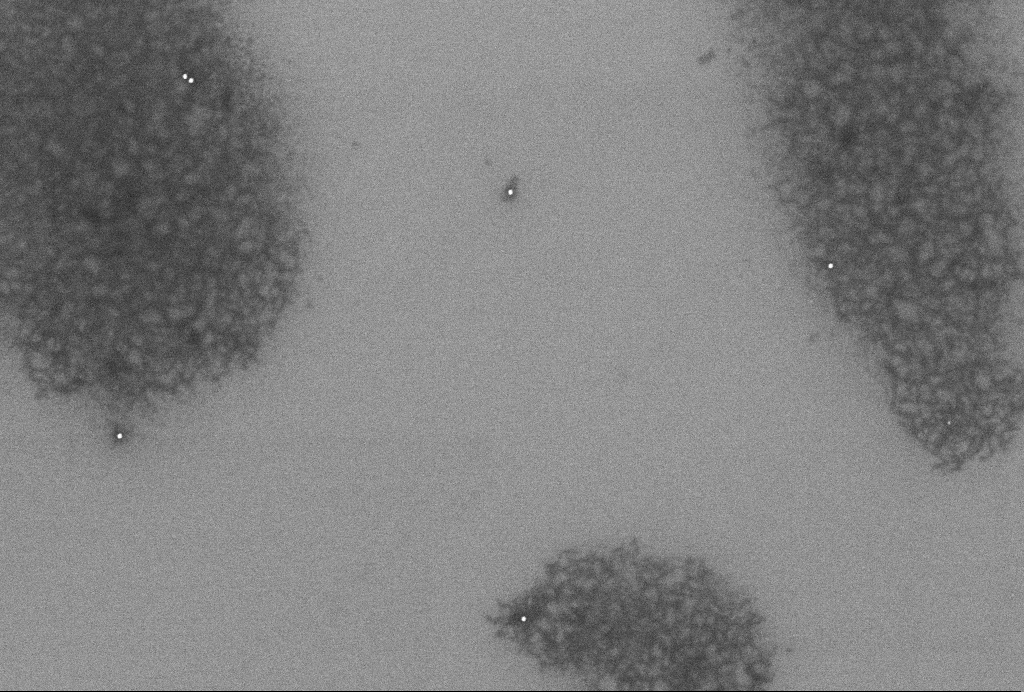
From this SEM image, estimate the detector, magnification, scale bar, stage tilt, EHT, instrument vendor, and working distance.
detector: InLens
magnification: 58.32 K X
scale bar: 1000 nm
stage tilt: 0°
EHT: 2 kV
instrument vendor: Zeiss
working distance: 3.3 mm